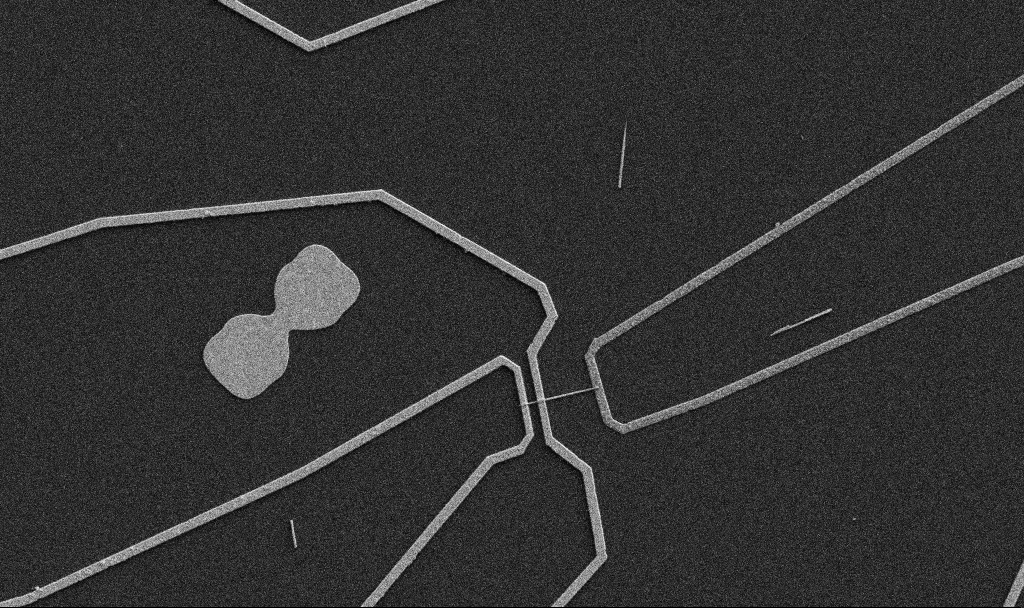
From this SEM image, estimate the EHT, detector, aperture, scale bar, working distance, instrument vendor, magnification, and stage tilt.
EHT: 5 kV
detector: SE2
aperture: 30 µm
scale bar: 10000 nm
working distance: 10.7 mm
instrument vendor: Zeiss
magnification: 5 K X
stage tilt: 0°